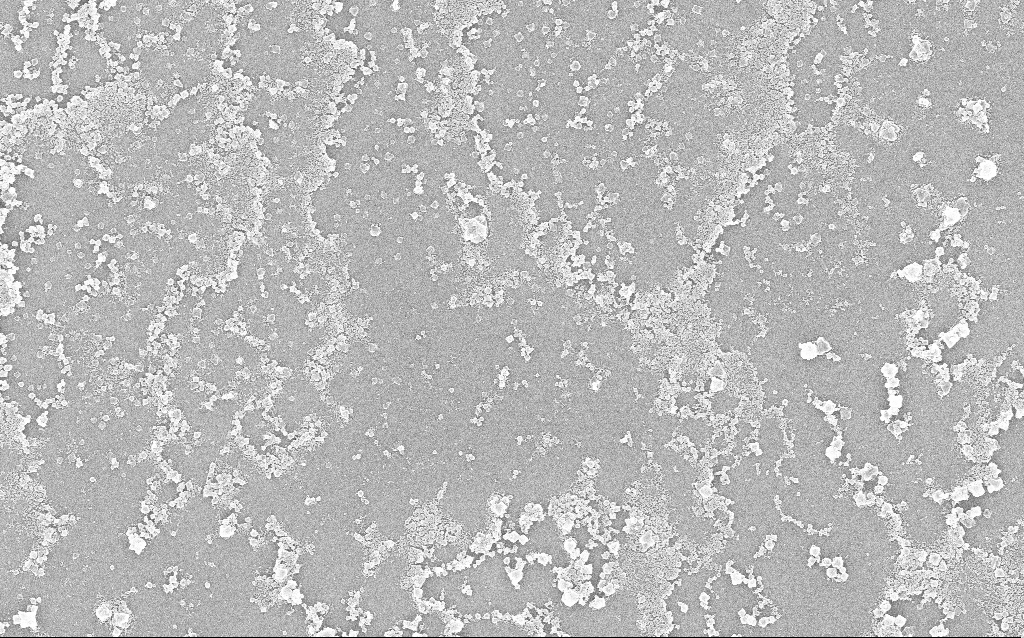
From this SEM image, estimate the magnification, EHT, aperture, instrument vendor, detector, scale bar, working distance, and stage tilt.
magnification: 10 K X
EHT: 20 kV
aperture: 30 µm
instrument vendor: Zeiss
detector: InLens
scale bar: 2000 nm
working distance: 1.9 mm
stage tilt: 0°